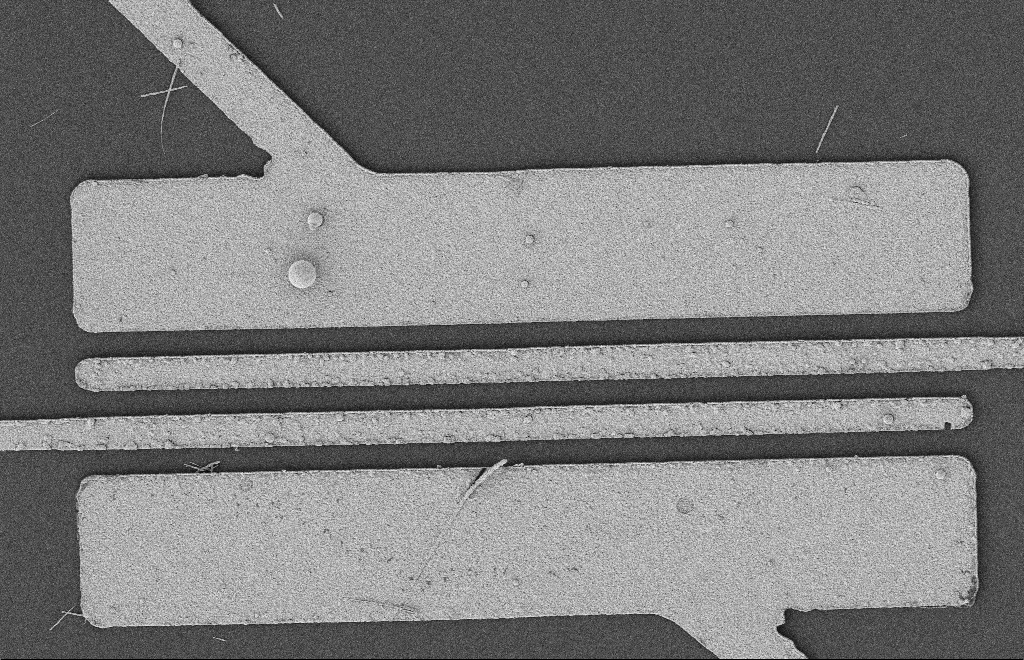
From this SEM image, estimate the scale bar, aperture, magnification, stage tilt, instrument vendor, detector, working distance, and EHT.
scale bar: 2000 nm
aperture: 20 µm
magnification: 5.37 K X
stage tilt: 0°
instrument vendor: Zeiss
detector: SE2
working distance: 12 mm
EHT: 2 kV